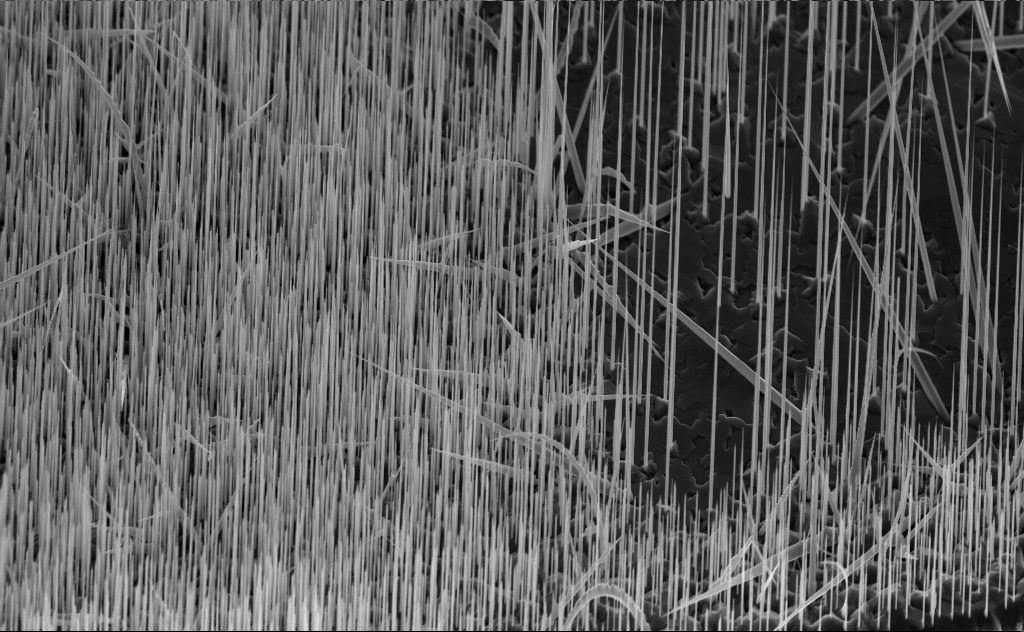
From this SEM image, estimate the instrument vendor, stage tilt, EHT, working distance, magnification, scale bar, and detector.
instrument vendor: Zeiss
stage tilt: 45°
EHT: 10 kV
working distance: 7 mm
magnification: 6.47 K X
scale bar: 10000 nm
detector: InLens